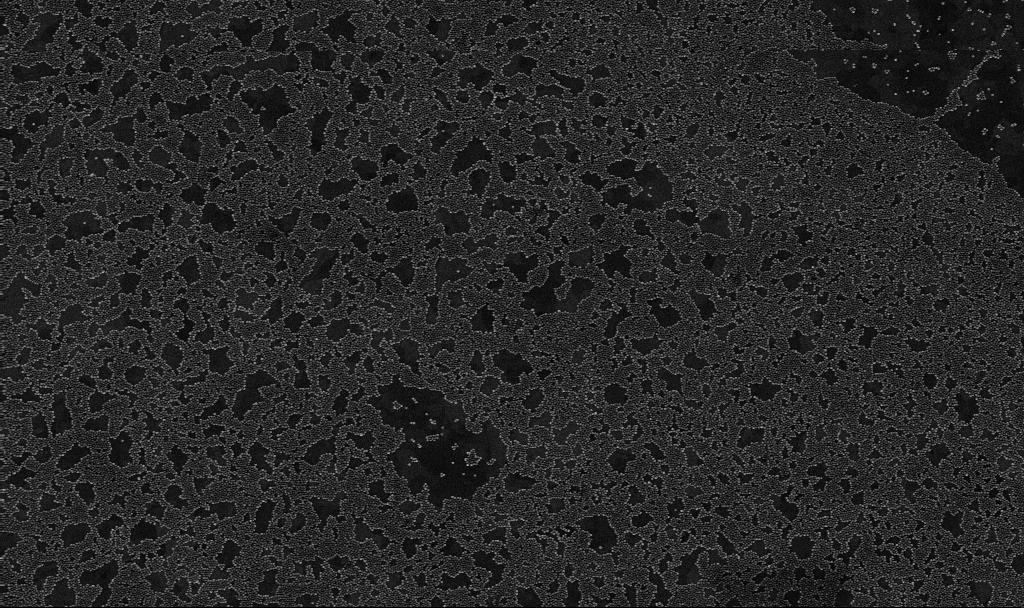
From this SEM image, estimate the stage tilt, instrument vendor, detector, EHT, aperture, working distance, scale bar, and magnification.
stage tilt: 0°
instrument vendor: Zeiss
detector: InLens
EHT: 10 kV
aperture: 30 µm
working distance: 3.4 mm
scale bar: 1000 nm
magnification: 50 K X